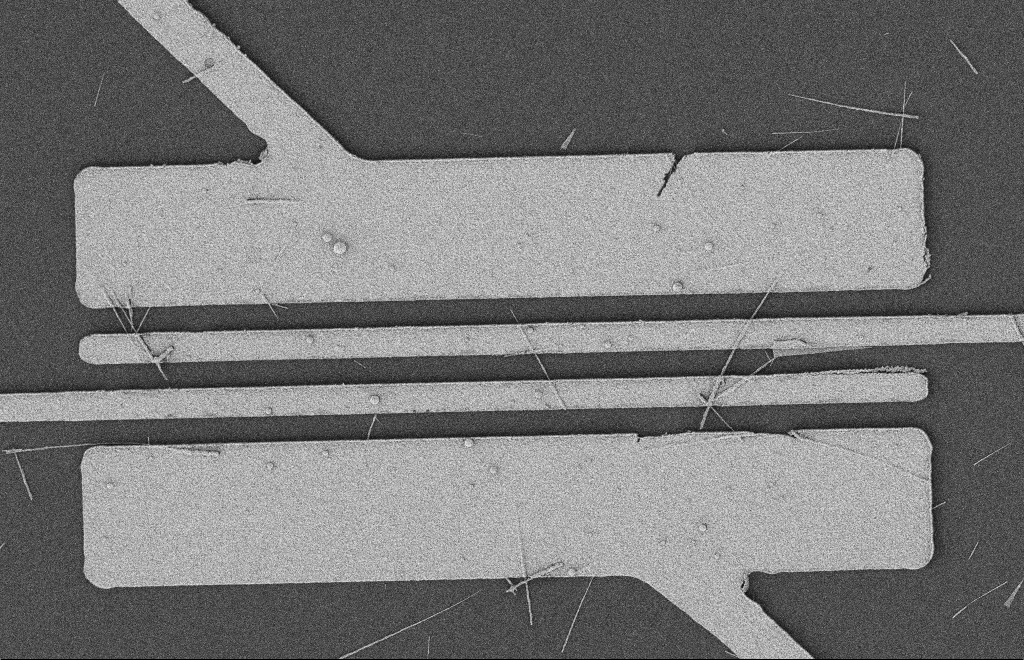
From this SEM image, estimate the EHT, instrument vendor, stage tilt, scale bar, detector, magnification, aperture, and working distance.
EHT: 2 kV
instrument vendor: Zeiss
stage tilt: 0°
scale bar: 2000 nm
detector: SE2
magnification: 5.1 K X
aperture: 20 µm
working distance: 12 mm